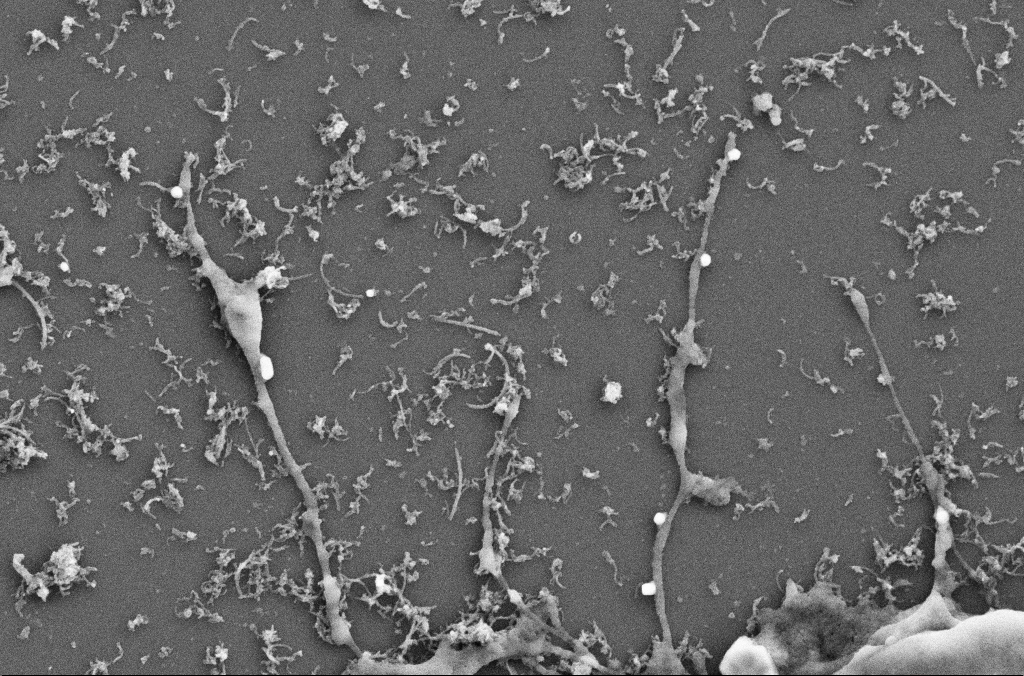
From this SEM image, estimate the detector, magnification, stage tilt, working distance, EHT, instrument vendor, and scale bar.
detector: SE2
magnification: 20 K X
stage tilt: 0°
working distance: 4 mm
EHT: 5 kV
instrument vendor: Zeiss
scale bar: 1000 nm